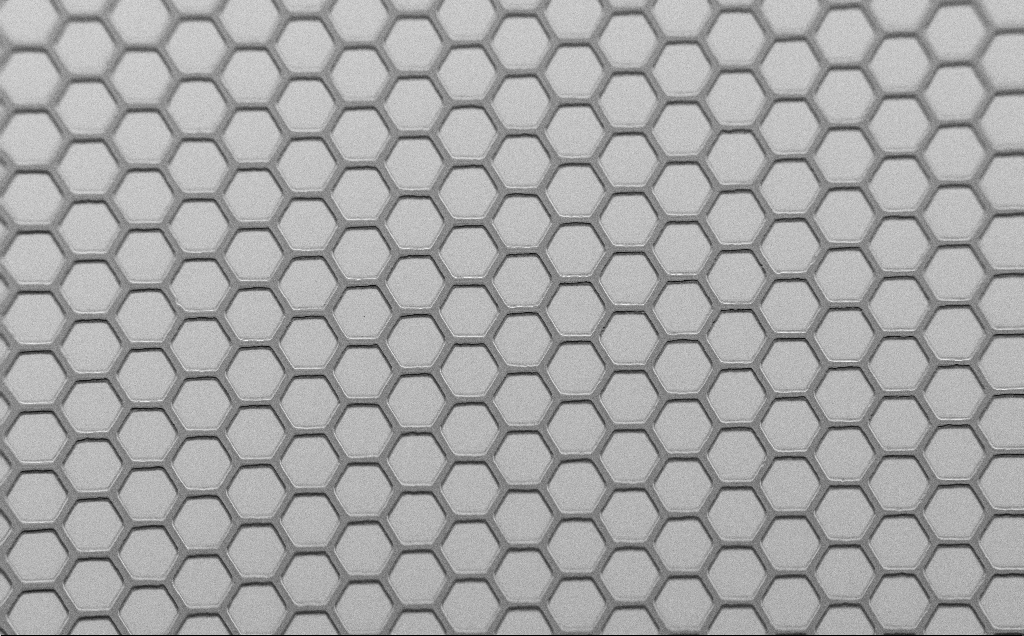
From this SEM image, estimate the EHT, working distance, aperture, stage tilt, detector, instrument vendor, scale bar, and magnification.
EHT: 1.5 kV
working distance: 7 mm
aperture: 30 µm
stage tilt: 45°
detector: SE2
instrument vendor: Zeiss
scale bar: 100000 nm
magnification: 0.375 K X